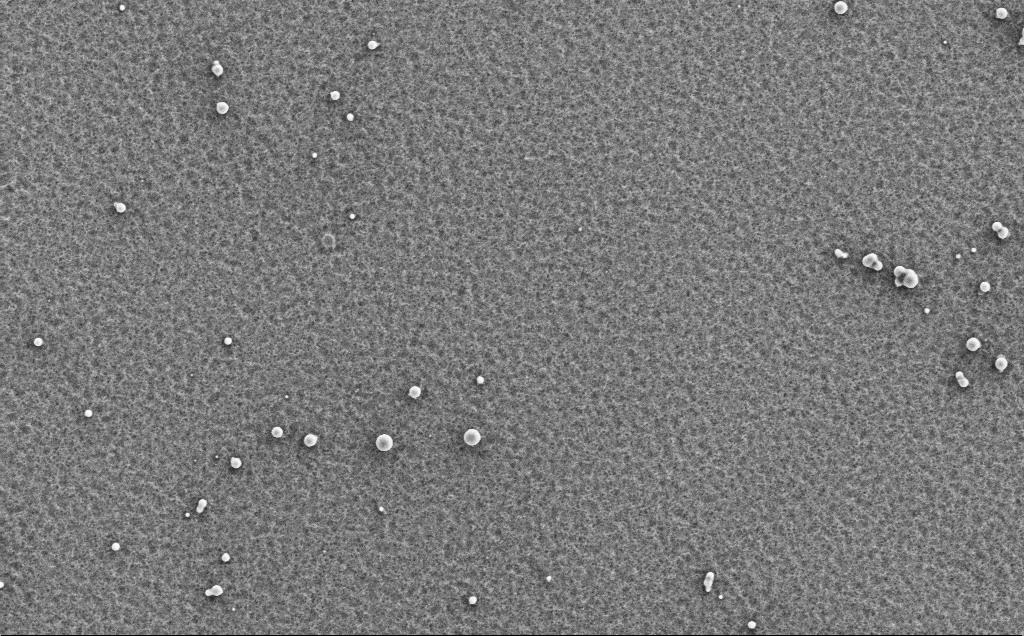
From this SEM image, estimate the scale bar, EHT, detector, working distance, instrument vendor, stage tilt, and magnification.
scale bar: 10000 nm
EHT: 5 kV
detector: SE2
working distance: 5 mm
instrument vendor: Zeiss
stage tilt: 0°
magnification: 4.14 K X